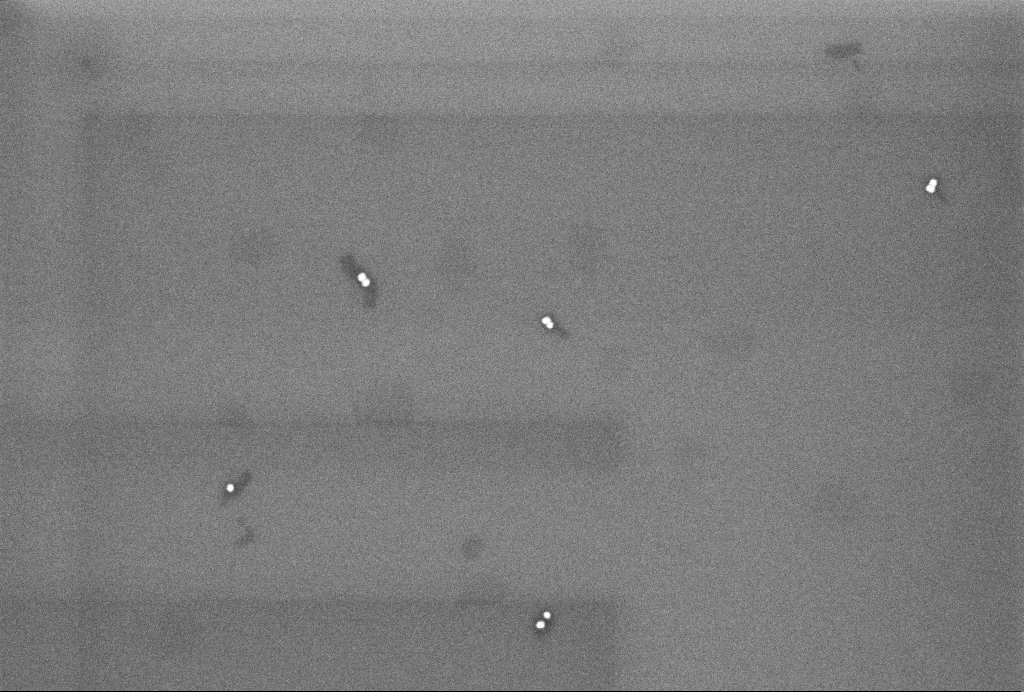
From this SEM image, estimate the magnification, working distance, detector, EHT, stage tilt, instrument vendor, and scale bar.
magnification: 104.21 K X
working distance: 3.2 mm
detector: InLens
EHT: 3 kV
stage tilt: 0°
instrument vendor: Zeiss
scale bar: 200 nm